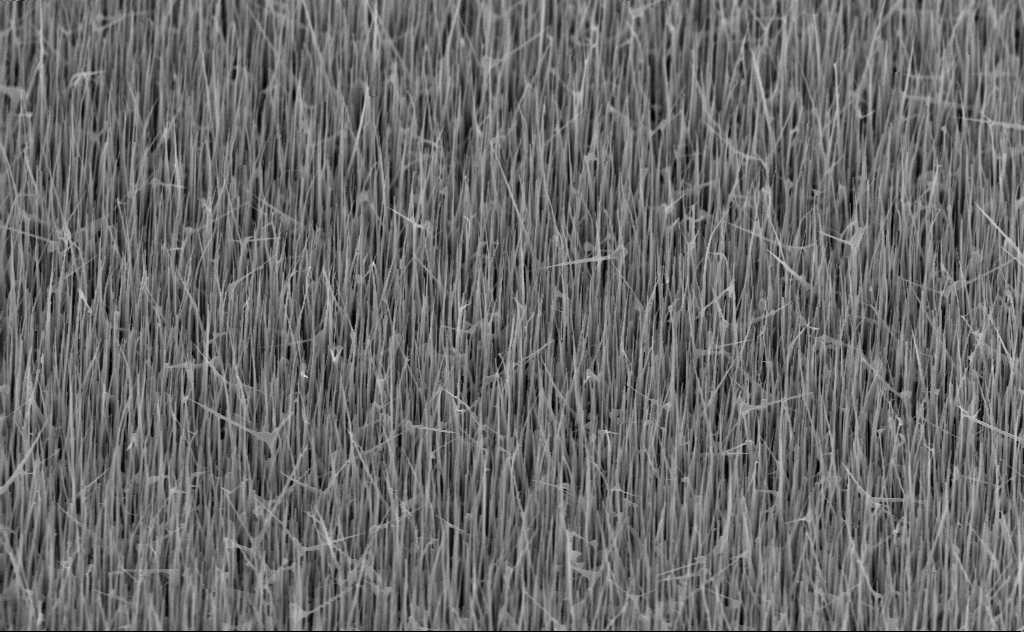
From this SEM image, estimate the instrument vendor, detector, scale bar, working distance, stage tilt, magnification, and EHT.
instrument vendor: Zeiss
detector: InLens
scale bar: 2000 nm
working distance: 6 mm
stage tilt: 45°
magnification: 20 K X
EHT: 10 kV